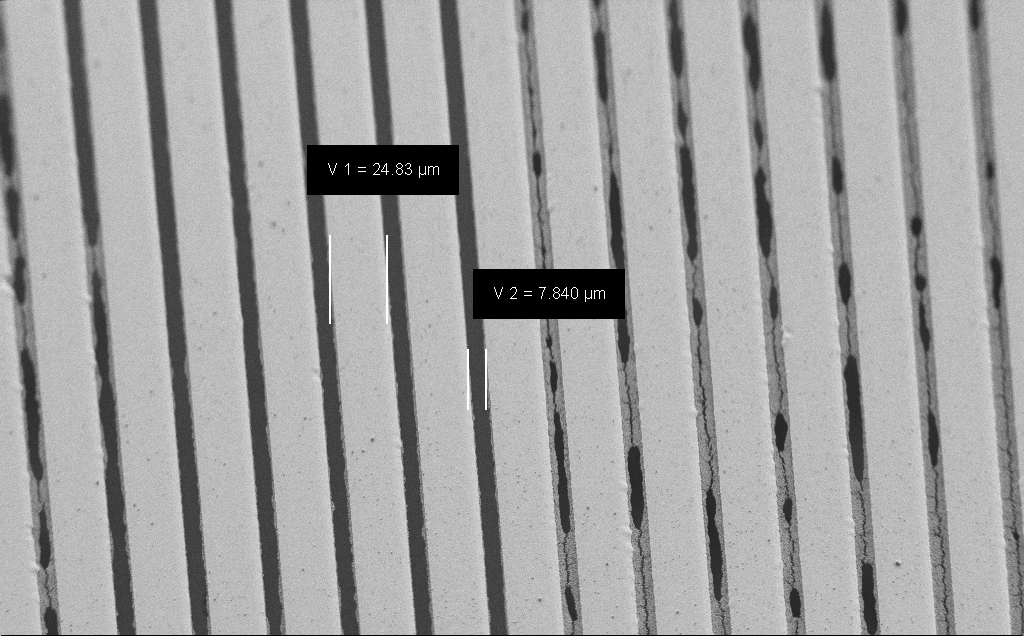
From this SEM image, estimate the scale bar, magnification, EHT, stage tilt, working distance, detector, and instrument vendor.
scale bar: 20000 nm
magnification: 0.843 K X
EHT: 1.2 kV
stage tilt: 45°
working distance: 7 mm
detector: SE2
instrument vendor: Zeiss